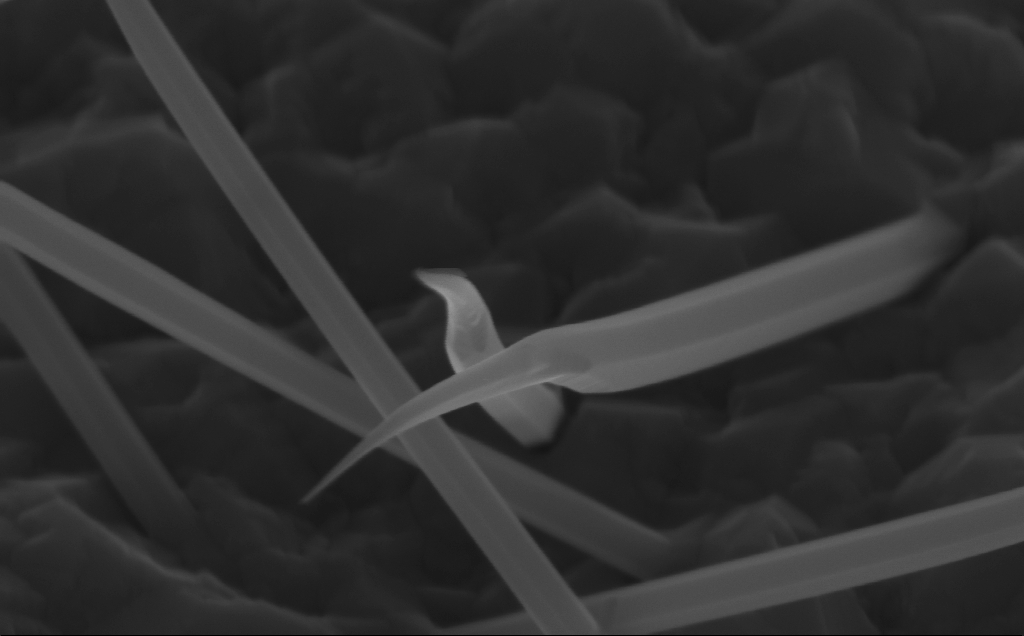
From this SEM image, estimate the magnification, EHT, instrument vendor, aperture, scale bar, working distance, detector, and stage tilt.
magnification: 175.69 K X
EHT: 10 kV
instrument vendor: Zeiss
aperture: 30 µm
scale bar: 200 nm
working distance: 5 mm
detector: InLens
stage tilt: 45°